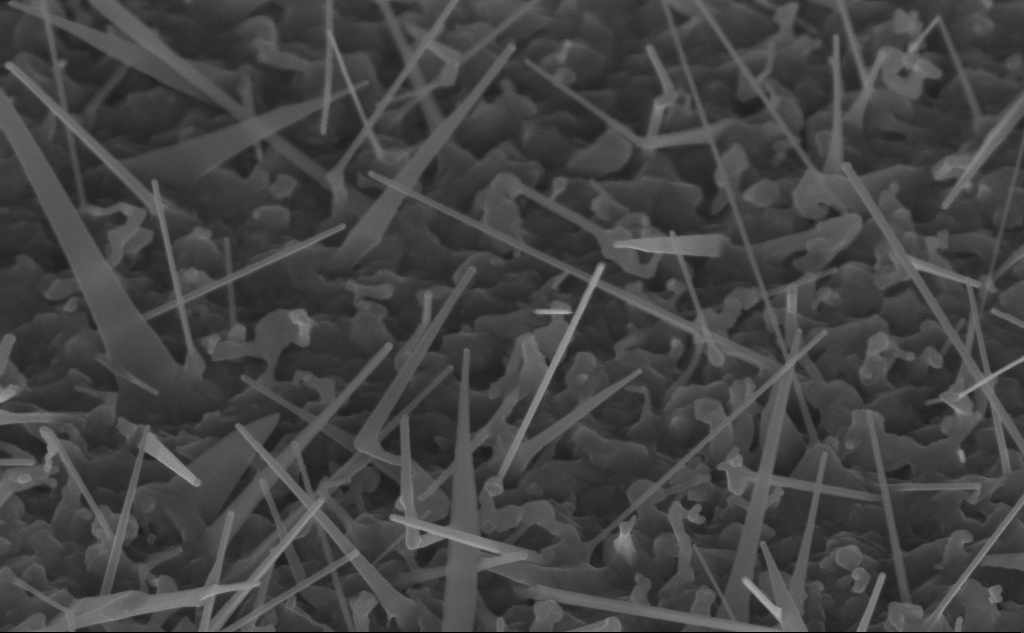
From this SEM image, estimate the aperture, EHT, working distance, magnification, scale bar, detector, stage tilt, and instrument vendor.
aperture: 30 µm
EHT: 10 kV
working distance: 8 mm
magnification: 80 K X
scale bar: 200 nm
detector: InLens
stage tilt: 45°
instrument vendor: Zeiss